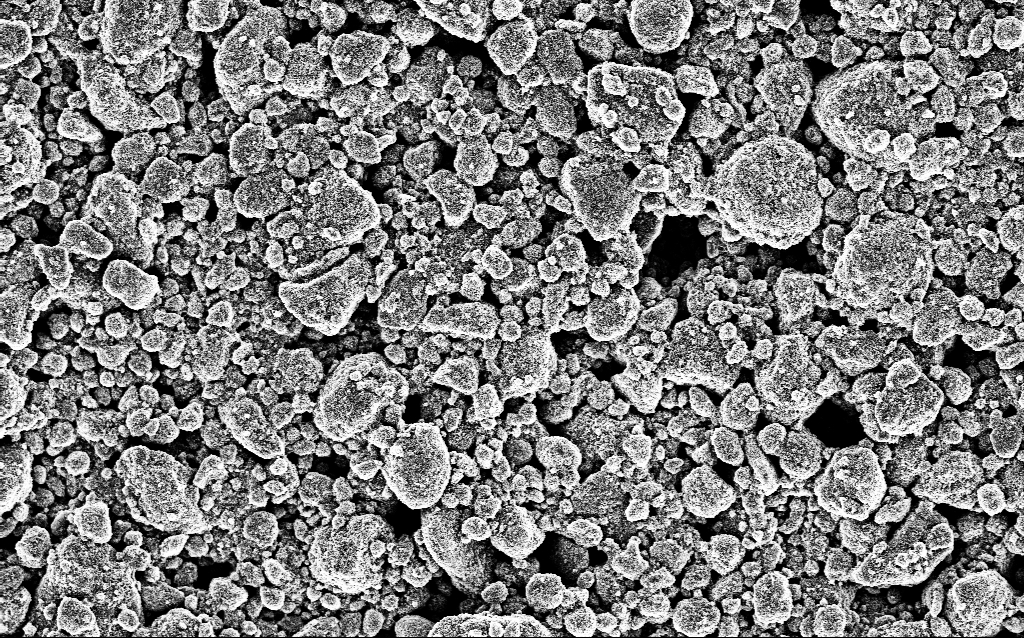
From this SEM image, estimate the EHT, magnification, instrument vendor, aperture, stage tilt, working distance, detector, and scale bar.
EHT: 5 kV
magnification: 4.52 K X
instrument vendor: Zeiss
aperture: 30 µm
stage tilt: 0°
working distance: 1.8 mm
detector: InLens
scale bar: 10000 nm